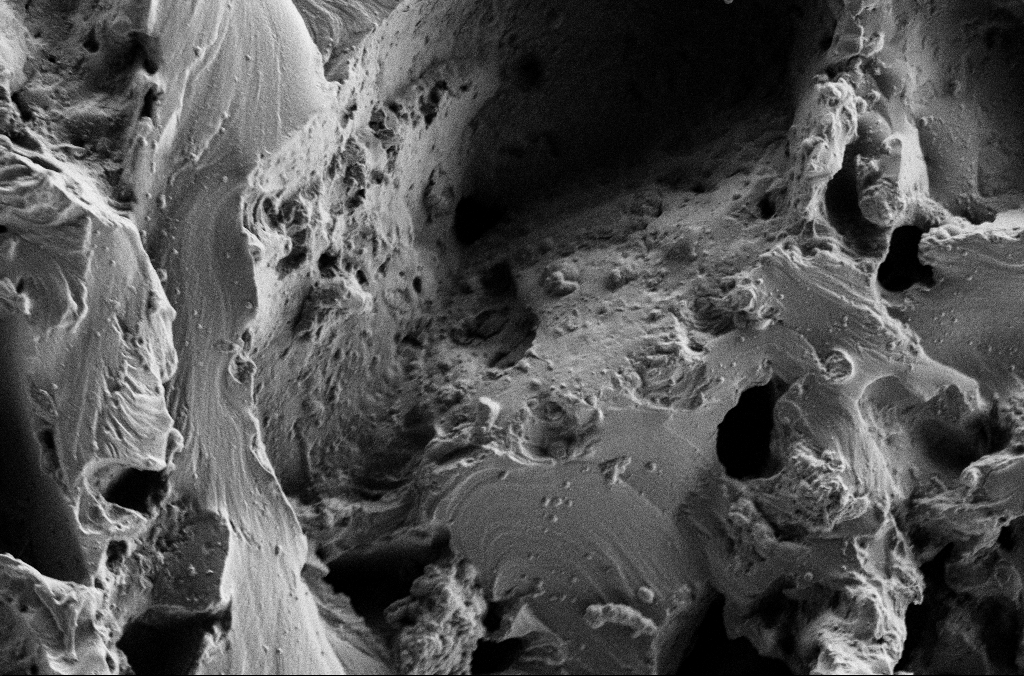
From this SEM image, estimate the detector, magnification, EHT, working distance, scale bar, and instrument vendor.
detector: SE2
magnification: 2.5 K X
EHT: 2 kV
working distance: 3 mm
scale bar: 10000 nm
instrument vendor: Zeiss